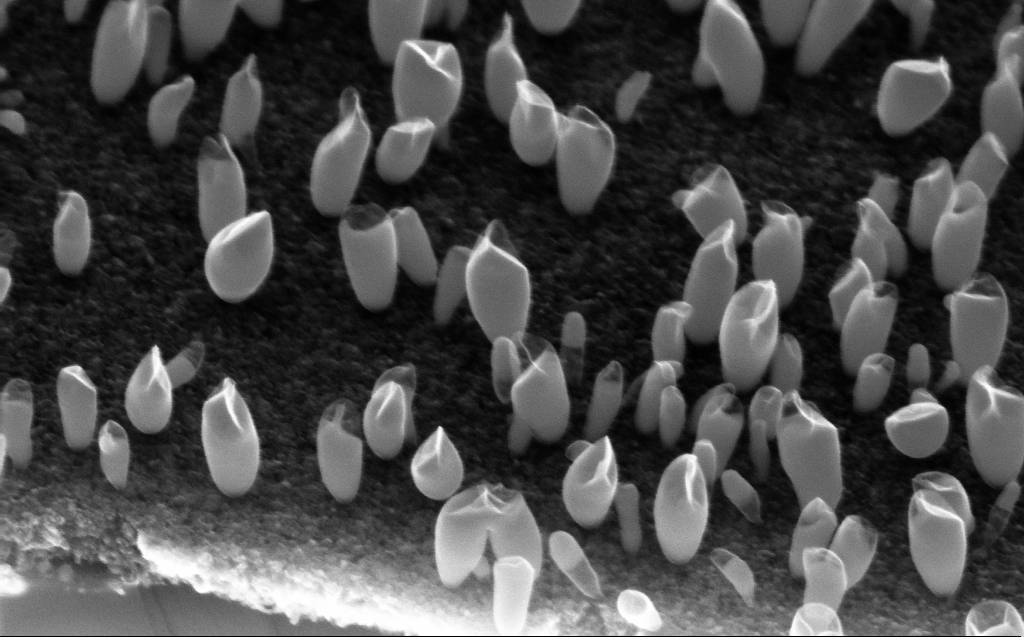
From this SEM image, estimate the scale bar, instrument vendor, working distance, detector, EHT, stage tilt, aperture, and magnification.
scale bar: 200 nm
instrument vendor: Zeiss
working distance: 5 mm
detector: InLens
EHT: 10 kV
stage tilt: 45°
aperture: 30 µm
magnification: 134.26 K X